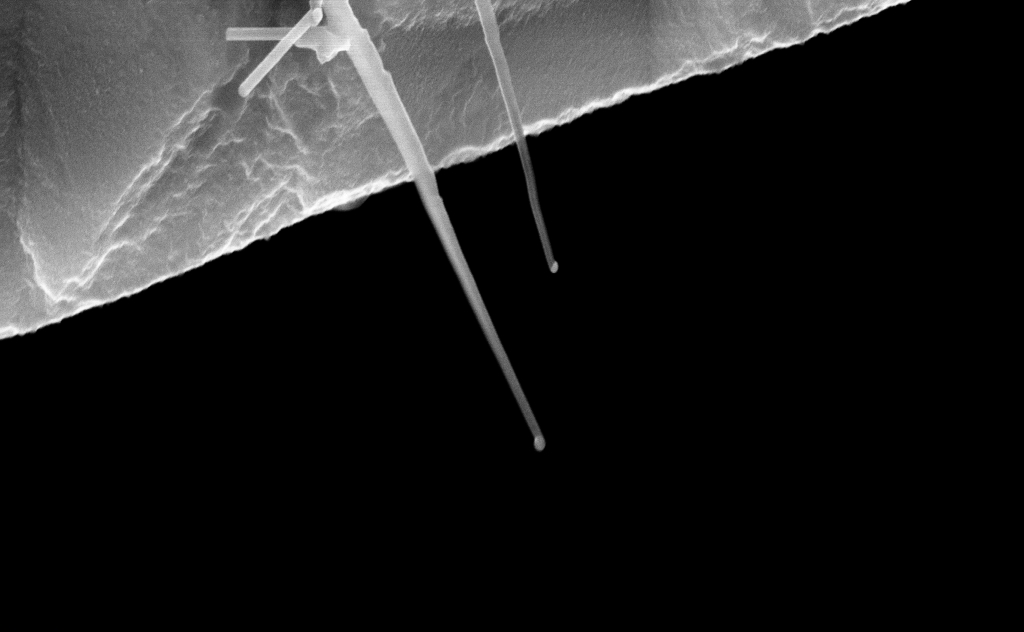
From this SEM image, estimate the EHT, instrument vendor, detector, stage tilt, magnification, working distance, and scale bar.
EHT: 20 kV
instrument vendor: Zeiss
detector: InLens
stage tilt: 0°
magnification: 69.05 K X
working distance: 8 mm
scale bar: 1000 nm